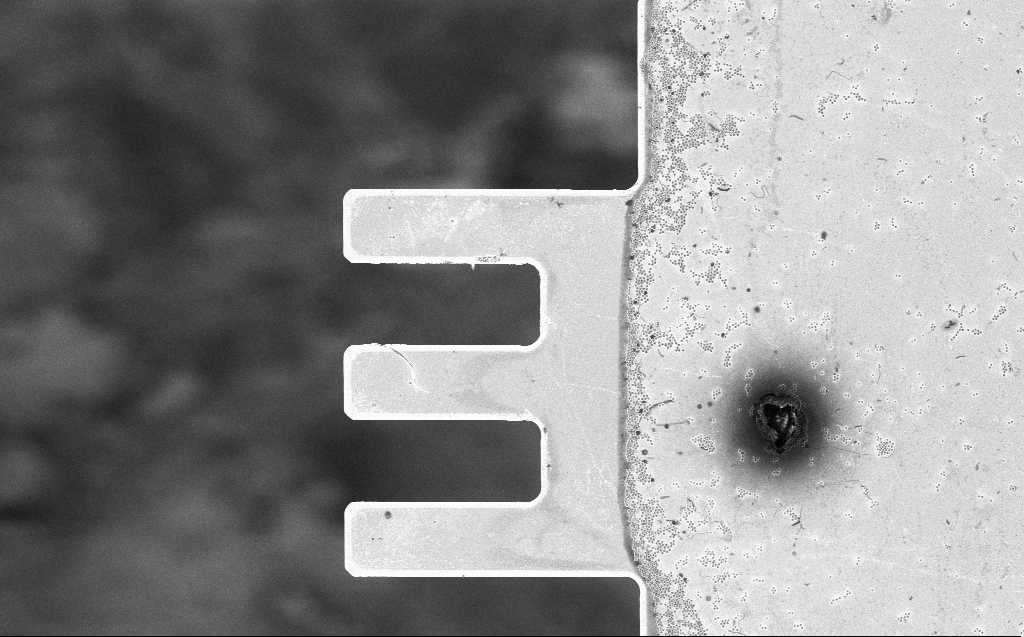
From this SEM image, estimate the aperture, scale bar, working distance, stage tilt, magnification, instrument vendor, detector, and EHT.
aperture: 30 µm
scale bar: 20000 nm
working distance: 7 mm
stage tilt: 0°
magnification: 1.44 K X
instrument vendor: Zeiss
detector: InLens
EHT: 3 kV